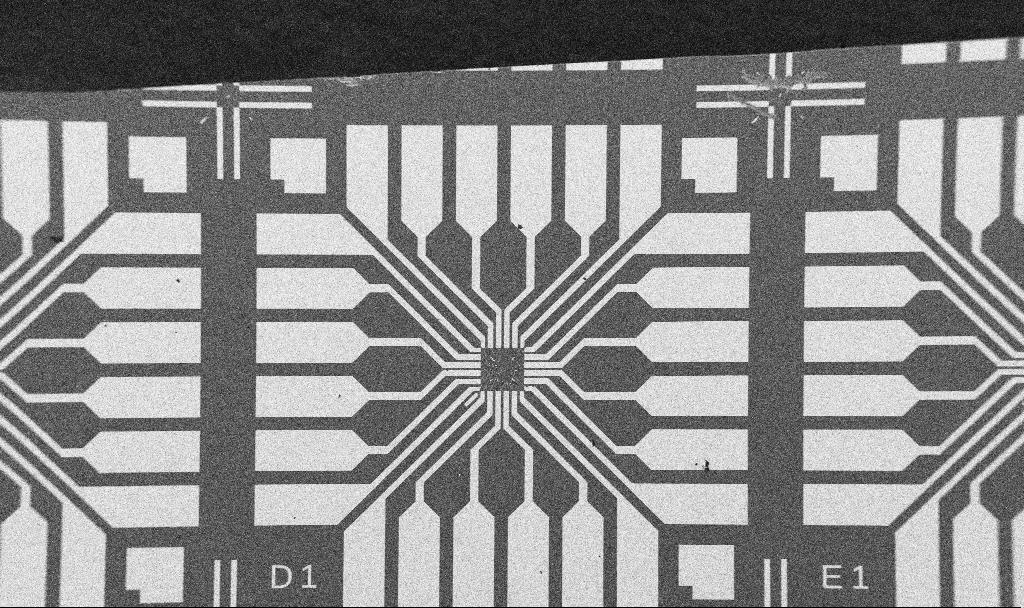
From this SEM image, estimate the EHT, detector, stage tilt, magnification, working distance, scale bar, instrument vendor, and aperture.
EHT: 5 kV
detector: SE2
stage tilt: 0°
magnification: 0.1 K X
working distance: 10.7 mm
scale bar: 200000 nm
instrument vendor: Zeiss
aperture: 30 µm